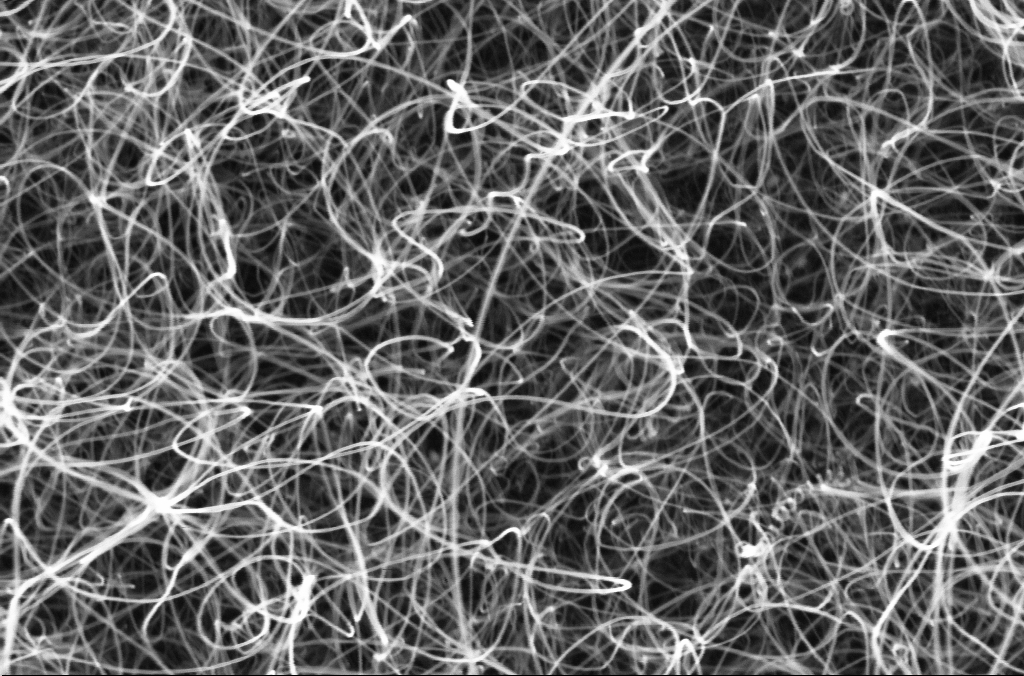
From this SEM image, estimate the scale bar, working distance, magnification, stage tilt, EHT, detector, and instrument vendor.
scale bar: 200 nm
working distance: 4.4 mm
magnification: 100 K X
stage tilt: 0°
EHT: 5 kV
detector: InLens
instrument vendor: Zeiss